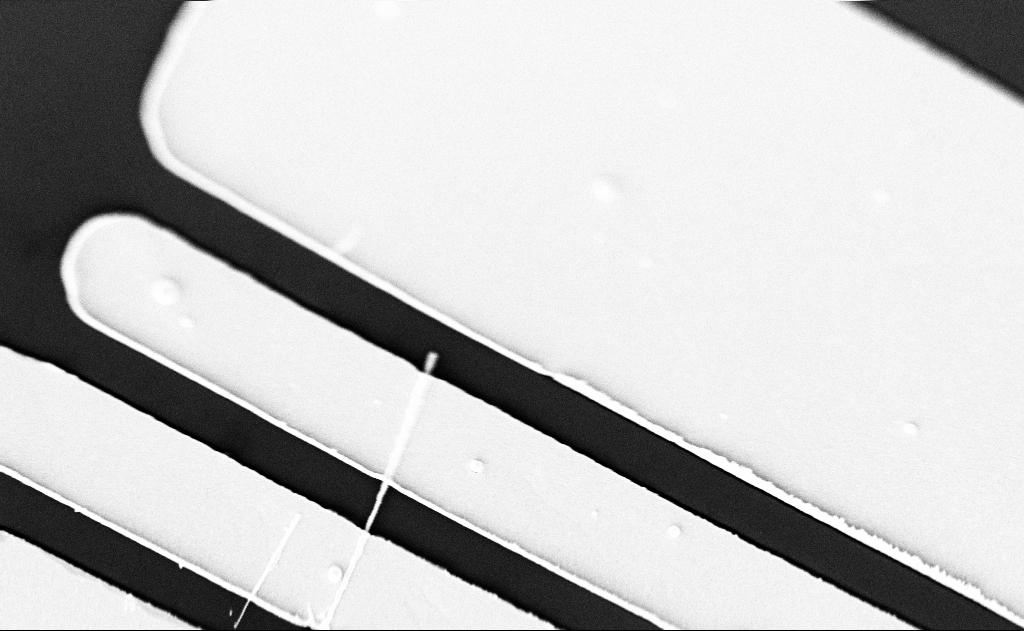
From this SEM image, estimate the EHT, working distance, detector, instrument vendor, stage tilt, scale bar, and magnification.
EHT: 5 kV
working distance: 20 mm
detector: SE2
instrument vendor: Zeiss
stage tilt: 0°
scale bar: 1000 nm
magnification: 15 K X